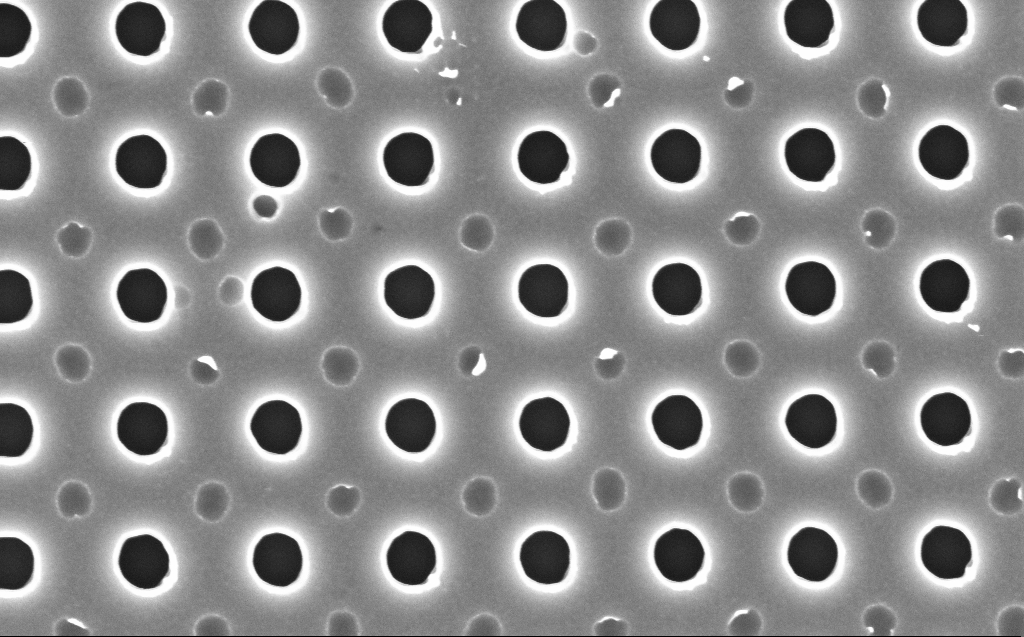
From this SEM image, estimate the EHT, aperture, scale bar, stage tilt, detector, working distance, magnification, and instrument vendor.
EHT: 5 kV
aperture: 30 µm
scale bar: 1000 nm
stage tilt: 0°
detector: InLens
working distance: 4 mm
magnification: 47.08 K X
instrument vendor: Zeiss